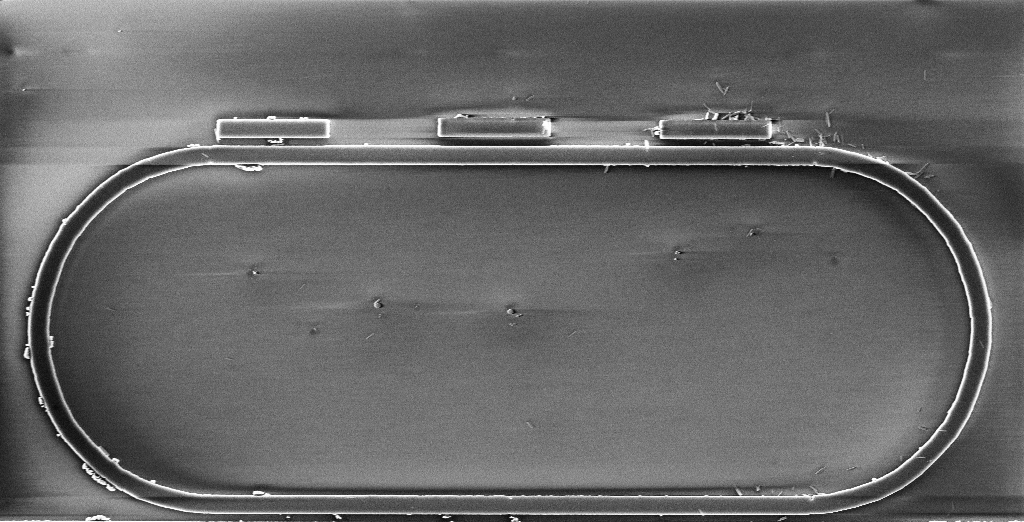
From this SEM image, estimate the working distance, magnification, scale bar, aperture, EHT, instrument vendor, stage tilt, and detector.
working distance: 3 mm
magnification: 13.71 K X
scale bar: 2000 nm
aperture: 30 µm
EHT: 3 kV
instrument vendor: Zeiss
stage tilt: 0°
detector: InLens